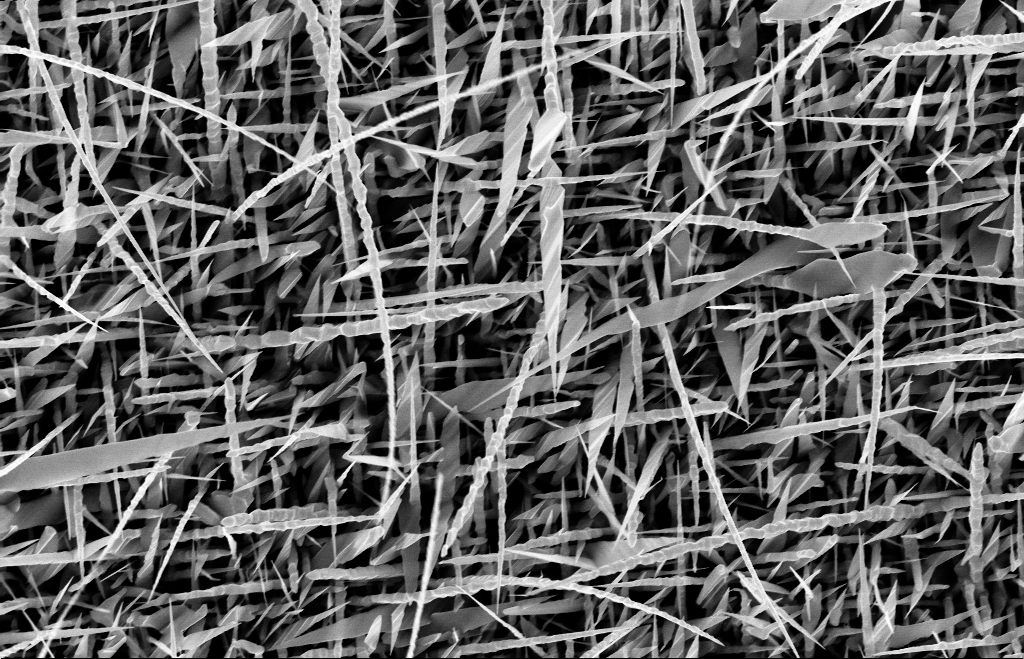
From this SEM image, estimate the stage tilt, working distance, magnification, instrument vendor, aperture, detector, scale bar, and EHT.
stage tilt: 0°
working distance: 9 mm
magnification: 20 K X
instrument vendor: Zeiss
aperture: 30 µm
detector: InLens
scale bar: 1000 nm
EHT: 10 kV